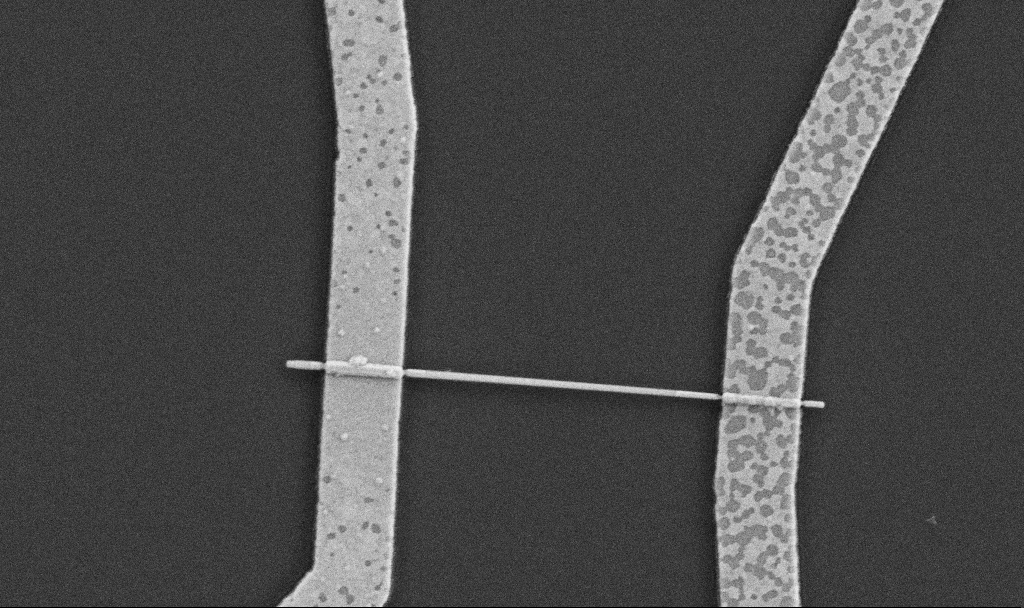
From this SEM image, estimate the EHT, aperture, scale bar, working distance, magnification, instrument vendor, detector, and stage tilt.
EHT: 5 kV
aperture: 30 µm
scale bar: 1000 nm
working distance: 8.7 mm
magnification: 30 K X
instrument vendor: Zeiss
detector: SE2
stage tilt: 0°